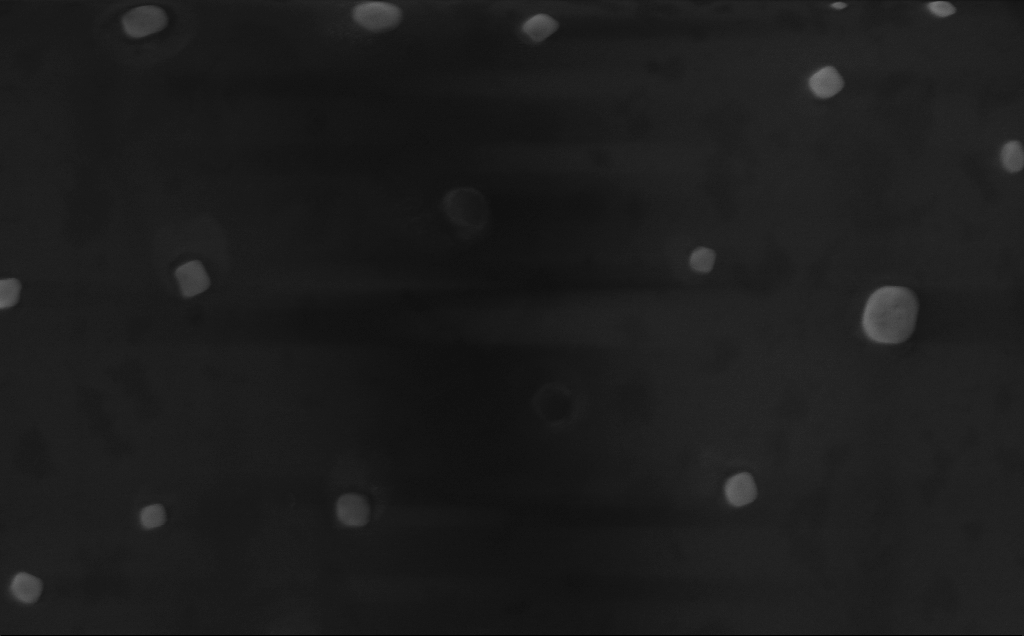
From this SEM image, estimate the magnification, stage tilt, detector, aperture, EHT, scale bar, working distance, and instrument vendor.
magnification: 77.54 K X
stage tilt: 0°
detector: InLens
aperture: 30 µm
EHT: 10 kV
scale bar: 200 nm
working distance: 5 mm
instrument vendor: Zeiss